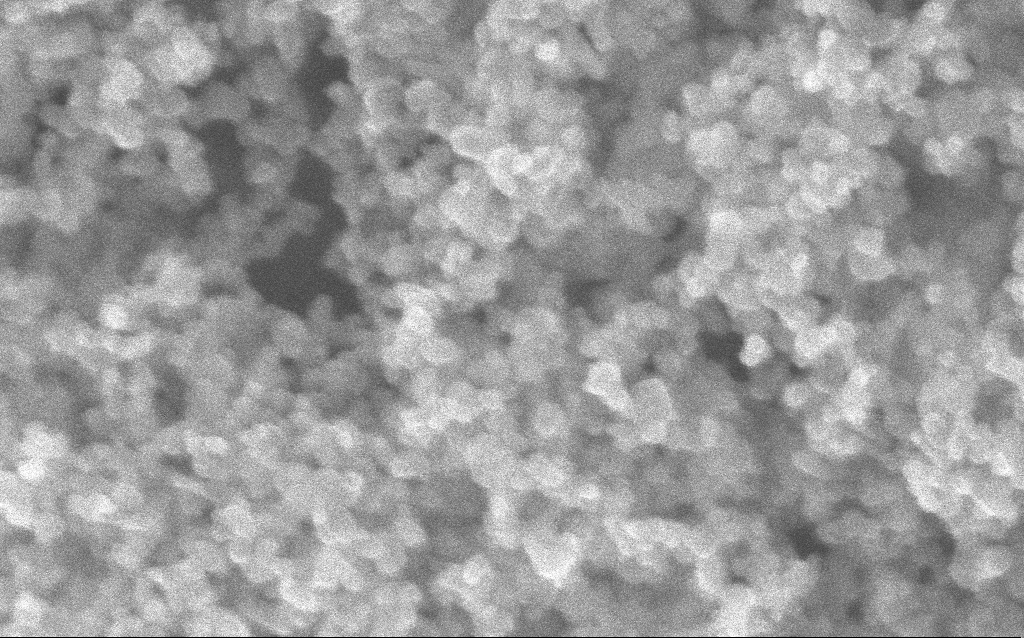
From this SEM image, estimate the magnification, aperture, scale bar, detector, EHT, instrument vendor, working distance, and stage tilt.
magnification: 348.1 K X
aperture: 30 µm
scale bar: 100 nm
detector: InLens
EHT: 10 kV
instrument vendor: Zeiss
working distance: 2.5 mm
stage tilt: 0°